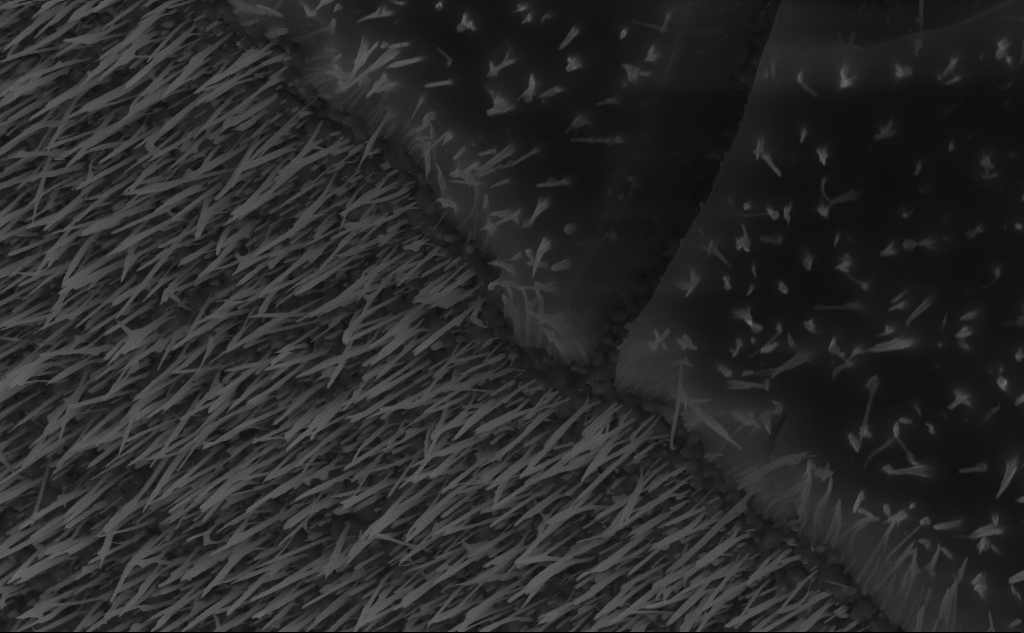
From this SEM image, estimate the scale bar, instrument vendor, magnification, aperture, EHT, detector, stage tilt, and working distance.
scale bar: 2000 nm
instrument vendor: Zeiss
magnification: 30.35 K X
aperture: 30 µm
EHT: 10 kV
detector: InLens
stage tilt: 45°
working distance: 6 mm